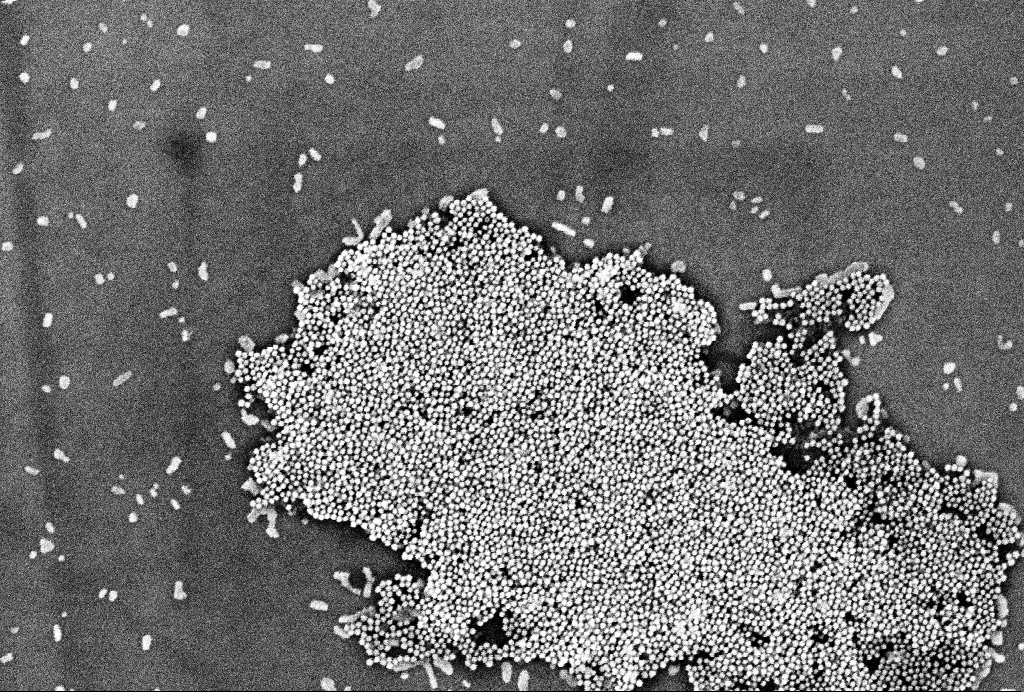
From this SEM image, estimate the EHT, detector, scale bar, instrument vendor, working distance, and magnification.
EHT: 2 kV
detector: InLens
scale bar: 200 nm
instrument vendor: Zeiss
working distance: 3.3 mm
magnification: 104.64 K X